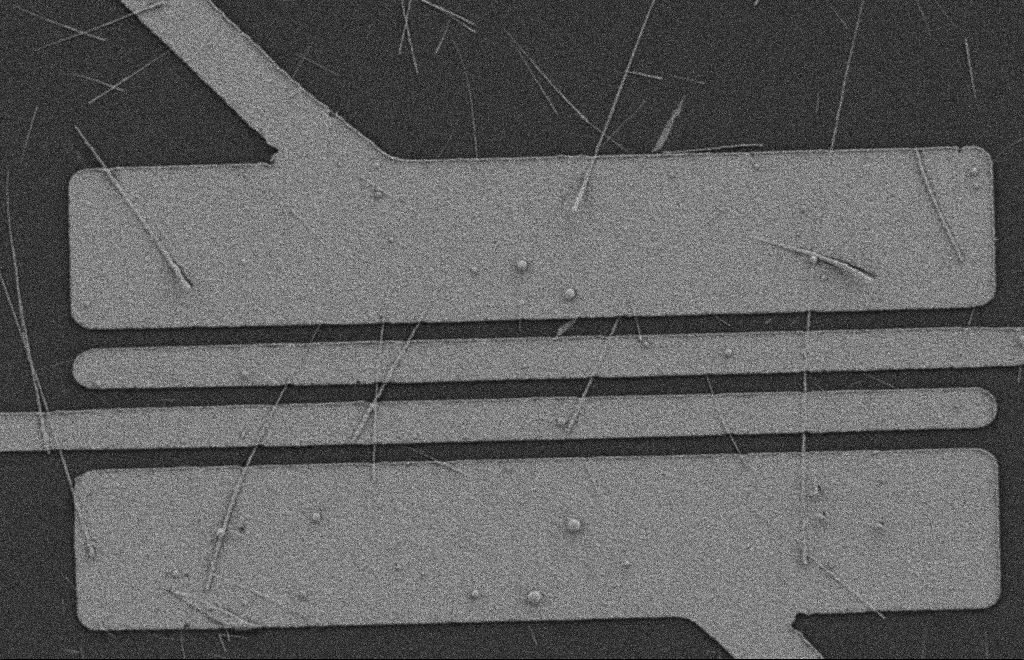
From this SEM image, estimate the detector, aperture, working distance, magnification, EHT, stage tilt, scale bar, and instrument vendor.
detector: SE2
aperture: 20 µm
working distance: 9 mm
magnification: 5.56 K X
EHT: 2 kV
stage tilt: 0°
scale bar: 2000 nm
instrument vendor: Zeiss